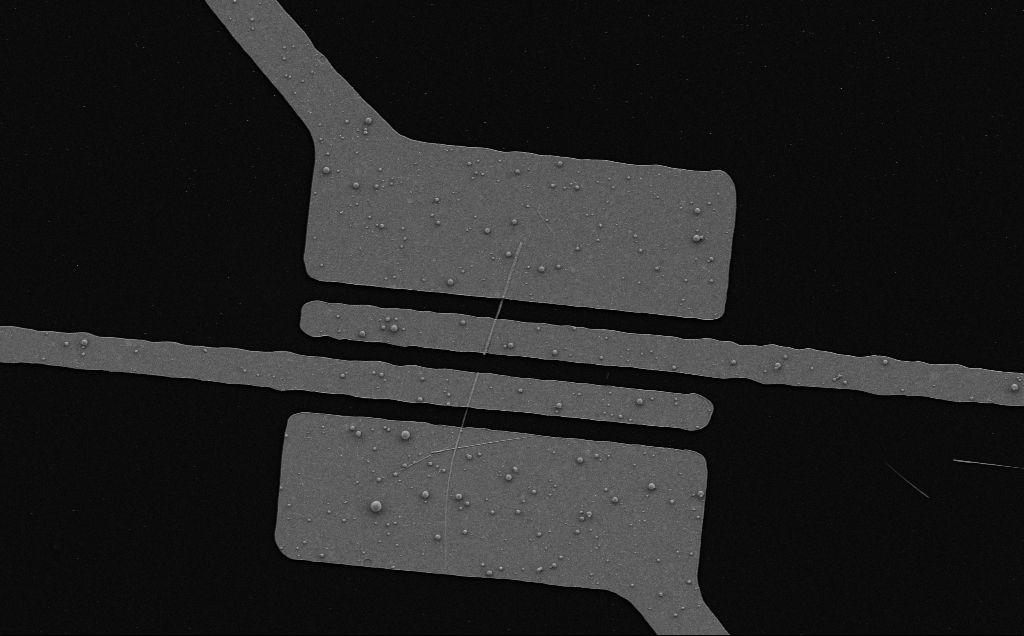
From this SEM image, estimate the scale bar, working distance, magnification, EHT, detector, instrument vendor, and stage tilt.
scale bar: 10000 nm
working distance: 8 mm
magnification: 5.13 K X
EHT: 5 kV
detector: SE2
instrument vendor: Zeiss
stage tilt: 0°